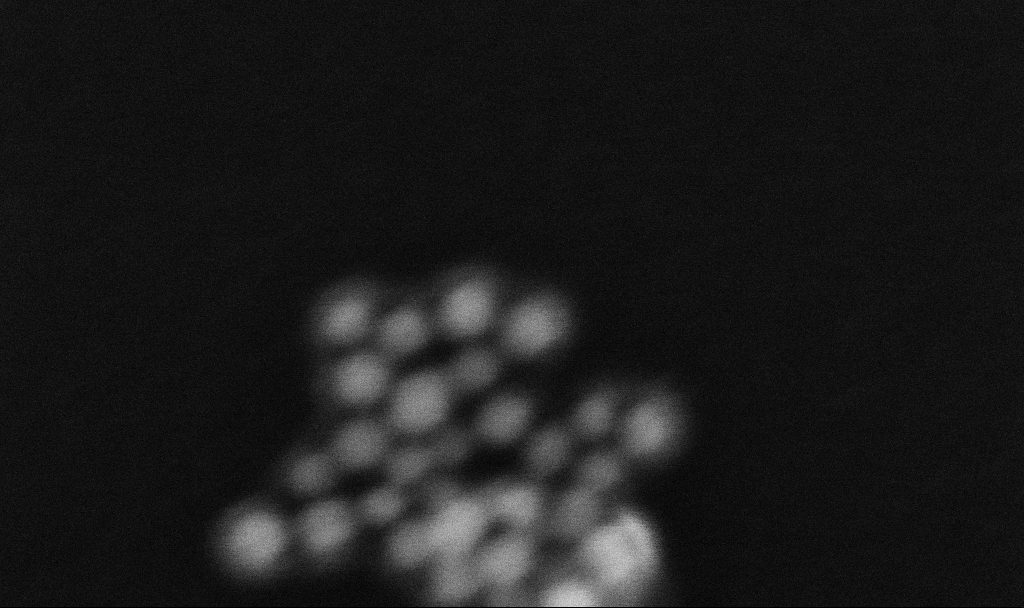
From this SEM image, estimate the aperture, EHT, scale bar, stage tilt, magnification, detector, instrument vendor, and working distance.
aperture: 30 µm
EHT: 10 kV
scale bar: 20 nm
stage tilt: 0°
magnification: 1000 K X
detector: InLens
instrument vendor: Zeiss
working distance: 3.3 mm